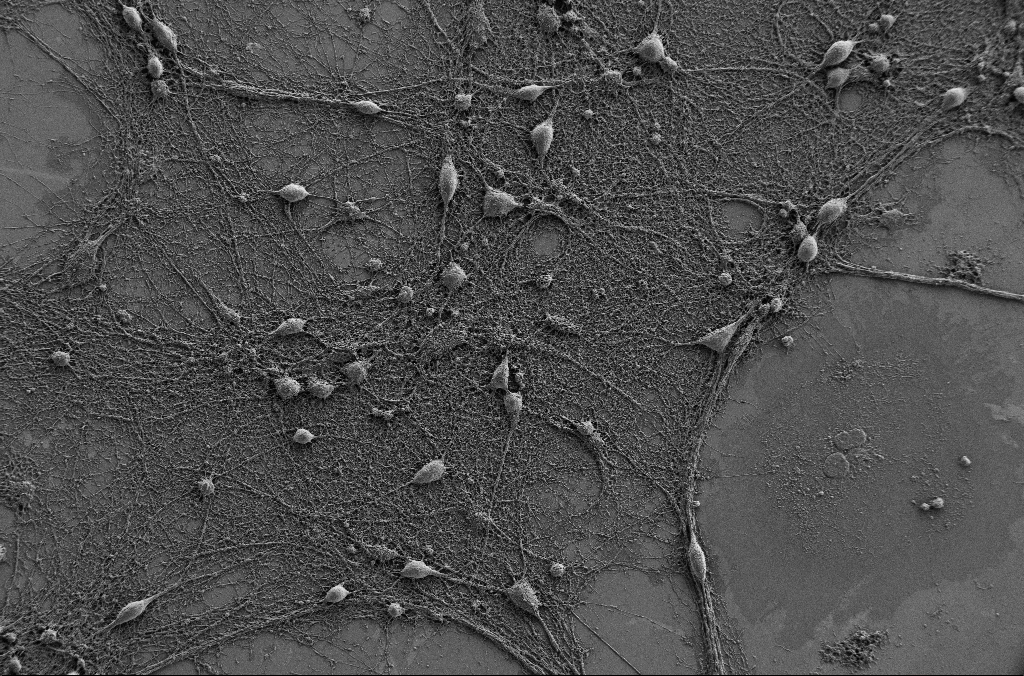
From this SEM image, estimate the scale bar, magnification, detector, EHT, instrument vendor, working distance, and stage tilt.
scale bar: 20000 nm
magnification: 1 K X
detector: SE2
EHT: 1 kV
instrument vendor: Zeiss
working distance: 4.1 mm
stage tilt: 0°